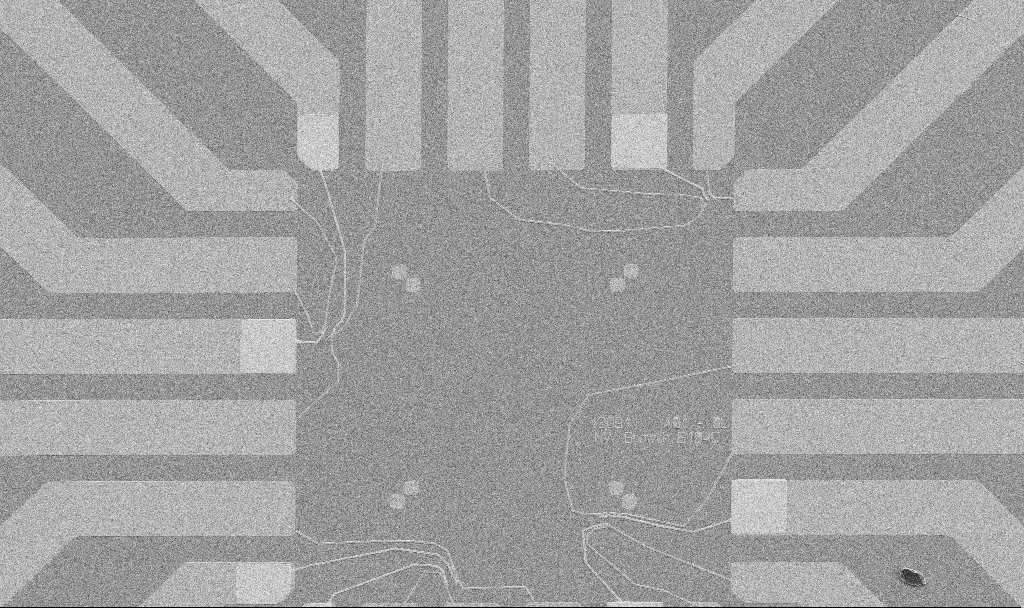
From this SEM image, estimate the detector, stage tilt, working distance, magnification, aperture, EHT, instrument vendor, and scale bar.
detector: SE2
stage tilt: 0°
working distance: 10.7 mm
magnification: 1 K X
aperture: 30 µm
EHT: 5 kV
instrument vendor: Zeiss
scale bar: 20000 nm